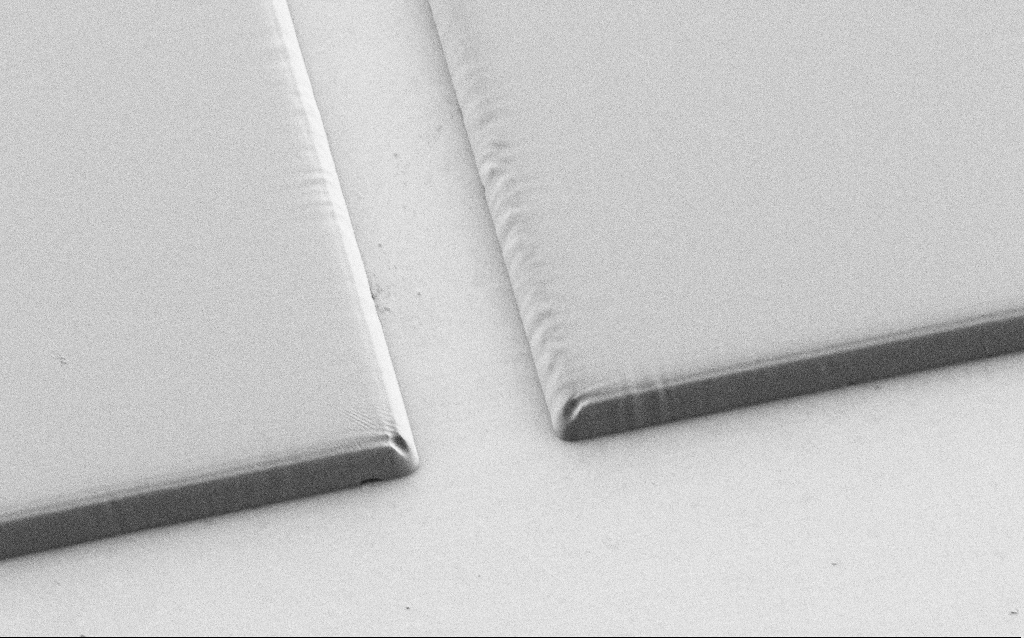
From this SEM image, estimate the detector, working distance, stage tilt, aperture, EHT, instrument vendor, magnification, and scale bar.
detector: SE2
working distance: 5 mm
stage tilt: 36°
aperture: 30 µm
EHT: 1 kV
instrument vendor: Zeiss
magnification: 2.66 K X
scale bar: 20000 nm